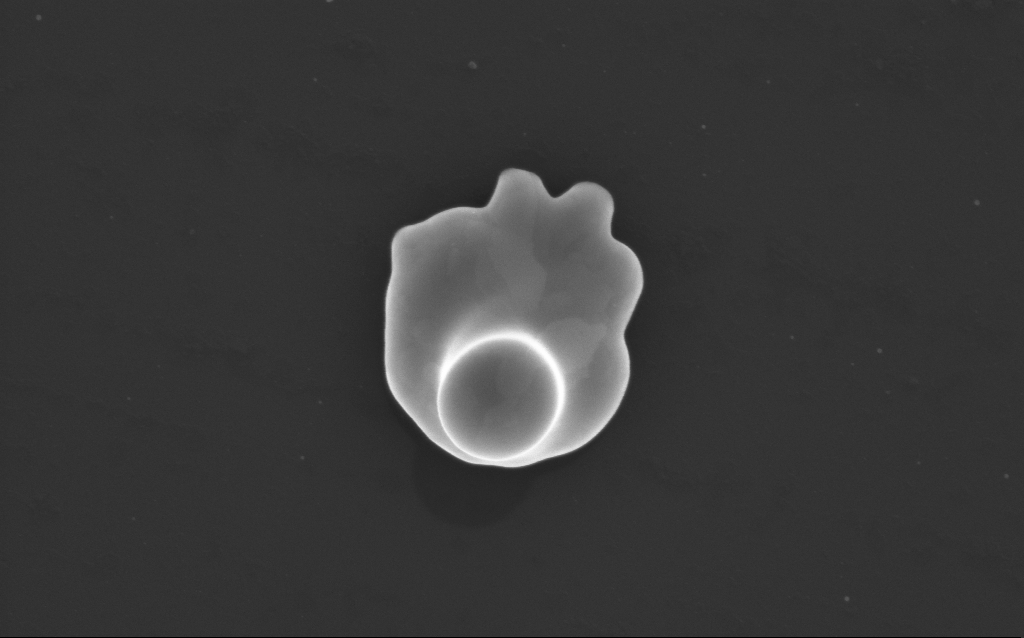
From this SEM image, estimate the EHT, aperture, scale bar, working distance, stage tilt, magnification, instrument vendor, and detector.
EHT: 10 kV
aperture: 30 µm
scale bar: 1000 nm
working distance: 3 mm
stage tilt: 0°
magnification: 66.94 K X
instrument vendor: Zeiss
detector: InLens